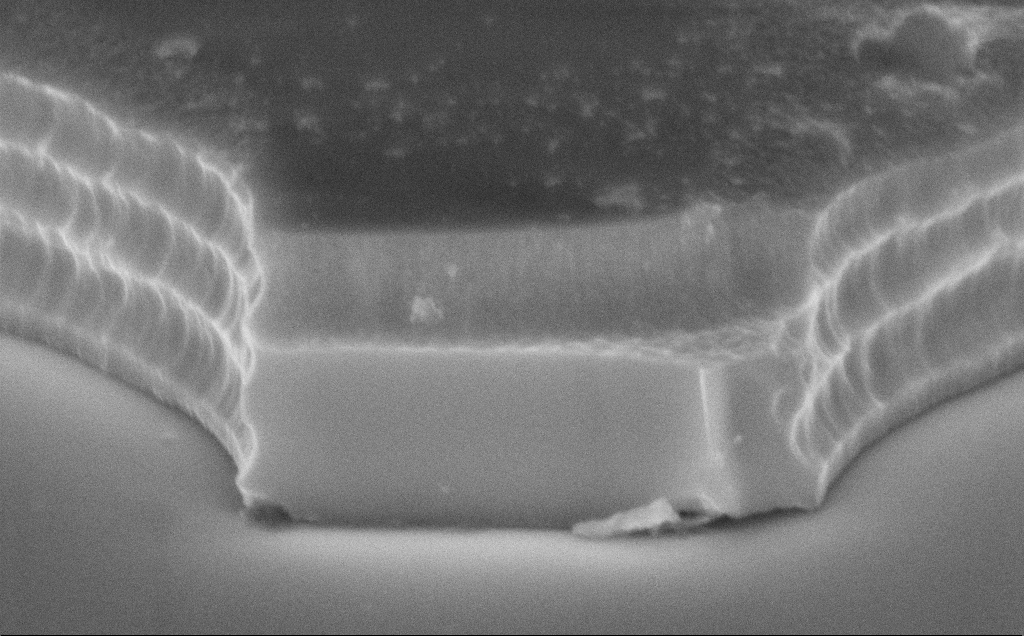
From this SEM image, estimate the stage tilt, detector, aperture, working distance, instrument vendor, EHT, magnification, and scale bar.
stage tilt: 70°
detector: InLens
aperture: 30 µm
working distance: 12 mm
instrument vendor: Zeiss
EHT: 8 kV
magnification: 42.09 K X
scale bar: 1000 nm